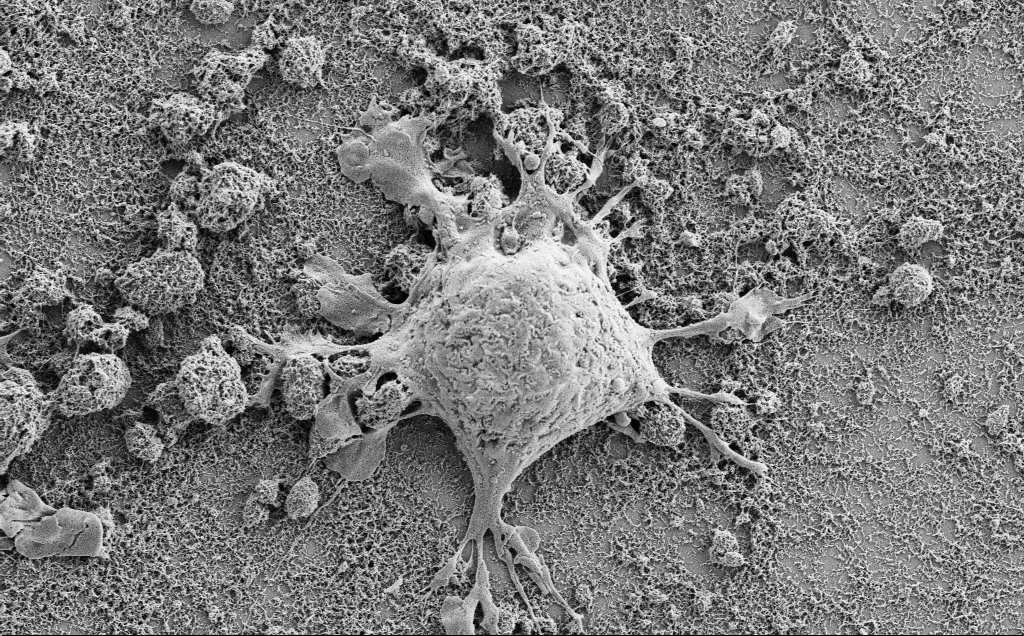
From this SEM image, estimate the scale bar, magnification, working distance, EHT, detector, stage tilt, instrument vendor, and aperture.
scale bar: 10000 nm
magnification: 7 K X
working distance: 7.1 mm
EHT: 2 kV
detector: SE2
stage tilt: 0°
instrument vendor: Zeiss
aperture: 30 µm